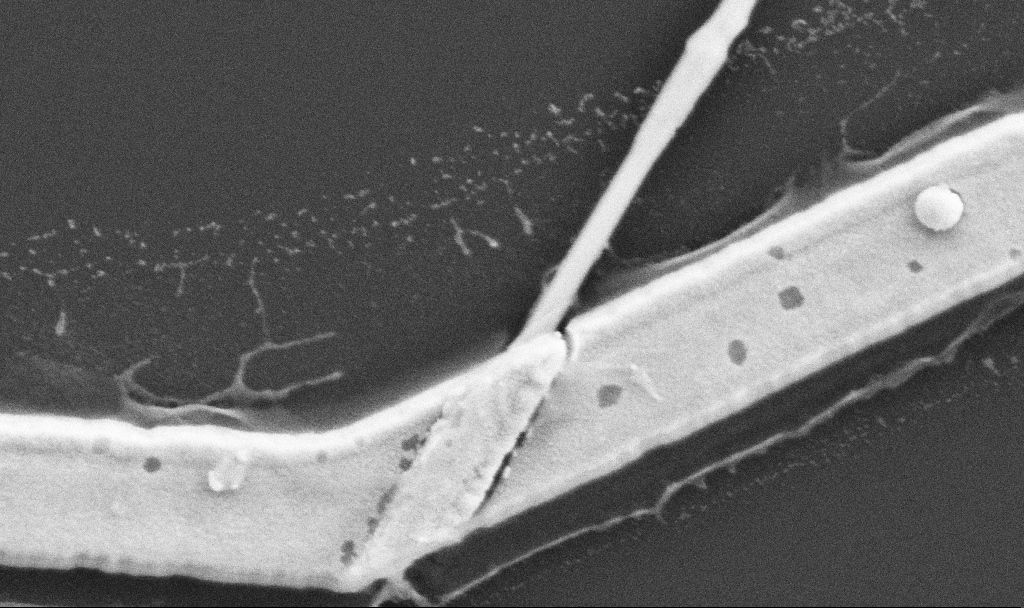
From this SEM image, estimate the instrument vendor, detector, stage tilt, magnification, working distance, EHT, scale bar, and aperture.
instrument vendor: Zeiss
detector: SE2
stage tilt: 0°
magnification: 80 K X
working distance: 10.7 mm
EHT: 5 kV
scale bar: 200 nm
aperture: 30 µm